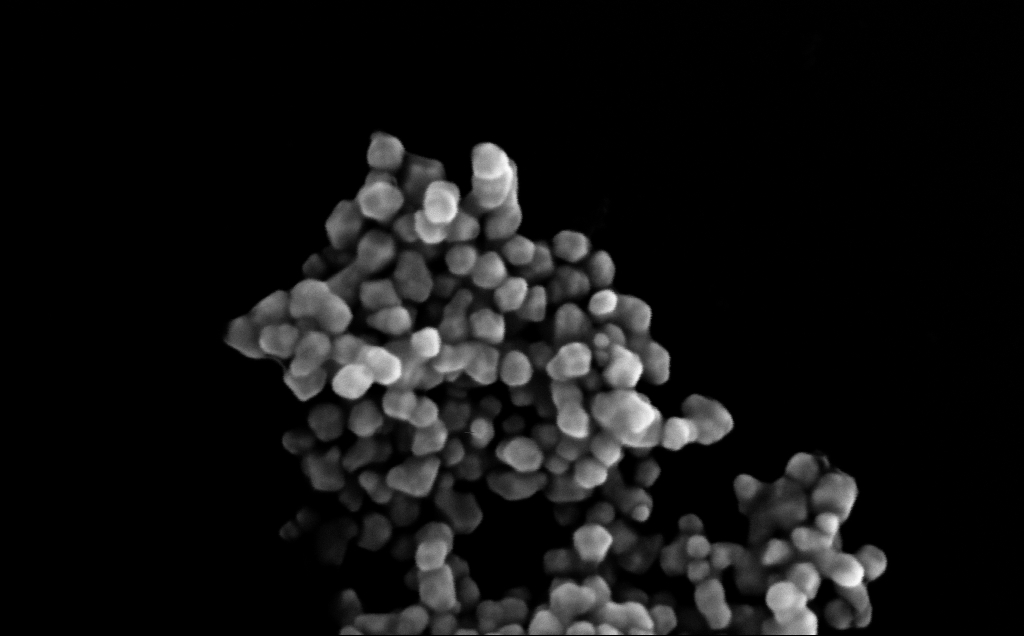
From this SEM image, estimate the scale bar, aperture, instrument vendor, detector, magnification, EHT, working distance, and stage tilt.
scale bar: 200 nm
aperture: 30 µm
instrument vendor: Zeiss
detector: InLens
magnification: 316.19 K X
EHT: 10 kV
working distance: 4 mm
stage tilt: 0°